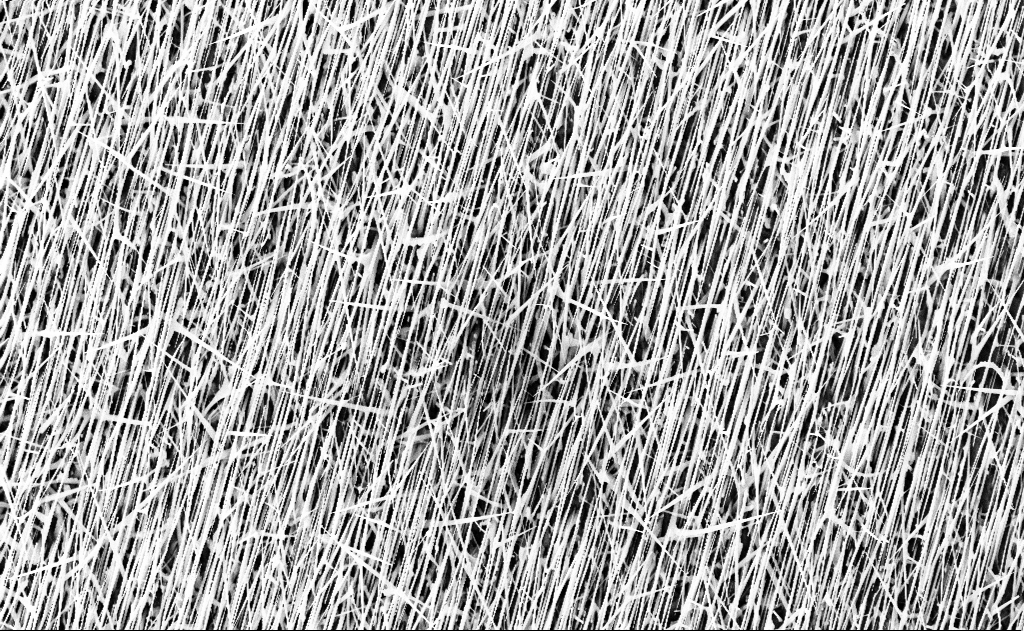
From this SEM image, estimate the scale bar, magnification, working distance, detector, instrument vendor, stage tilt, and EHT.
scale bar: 2000 nm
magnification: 10 K X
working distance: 16 mm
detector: InLens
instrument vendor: Zeiss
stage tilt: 0°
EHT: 10 kV